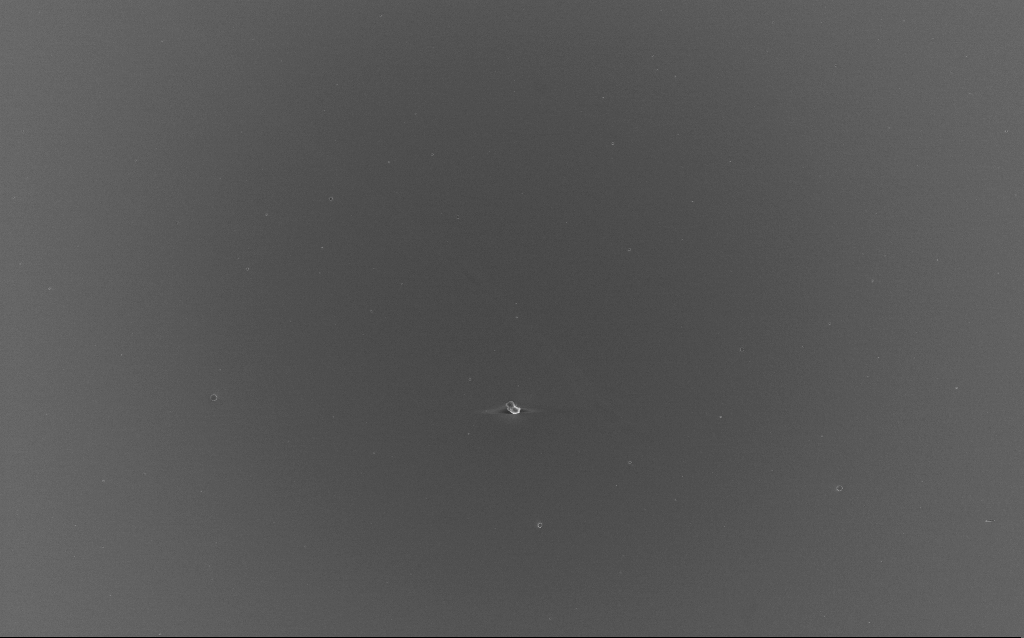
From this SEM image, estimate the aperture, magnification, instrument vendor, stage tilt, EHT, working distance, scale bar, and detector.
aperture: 30 µm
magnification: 0.604 K X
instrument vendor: Zeiss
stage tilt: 0°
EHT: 10 kV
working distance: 4 mm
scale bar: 100000 nm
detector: InLens